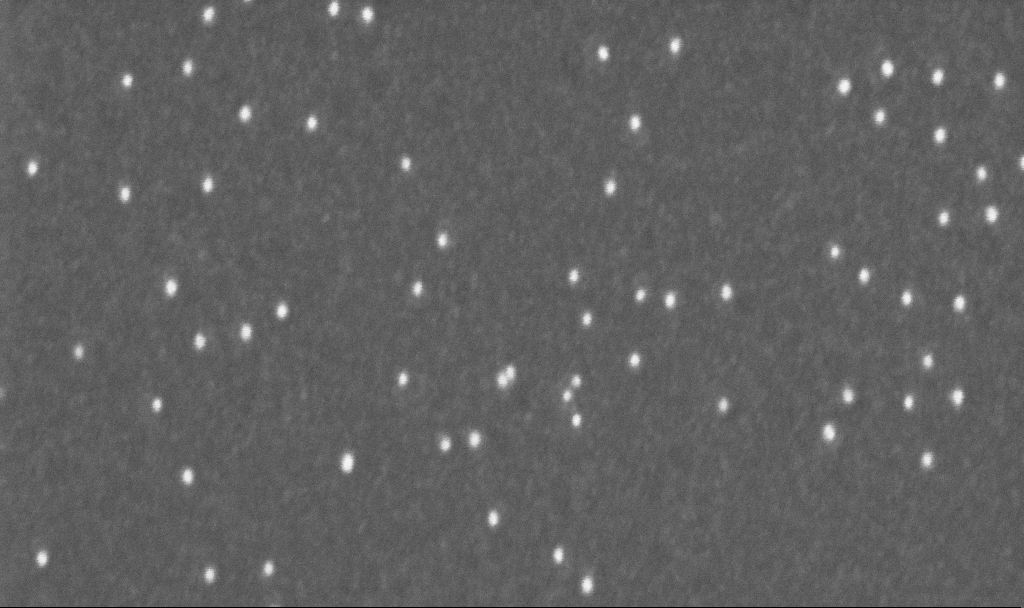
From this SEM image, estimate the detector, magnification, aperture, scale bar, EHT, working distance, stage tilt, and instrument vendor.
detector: InLens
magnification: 350 K X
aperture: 30 µm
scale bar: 200 nm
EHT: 10 kV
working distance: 3.2 mm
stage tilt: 0°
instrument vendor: Zeiss